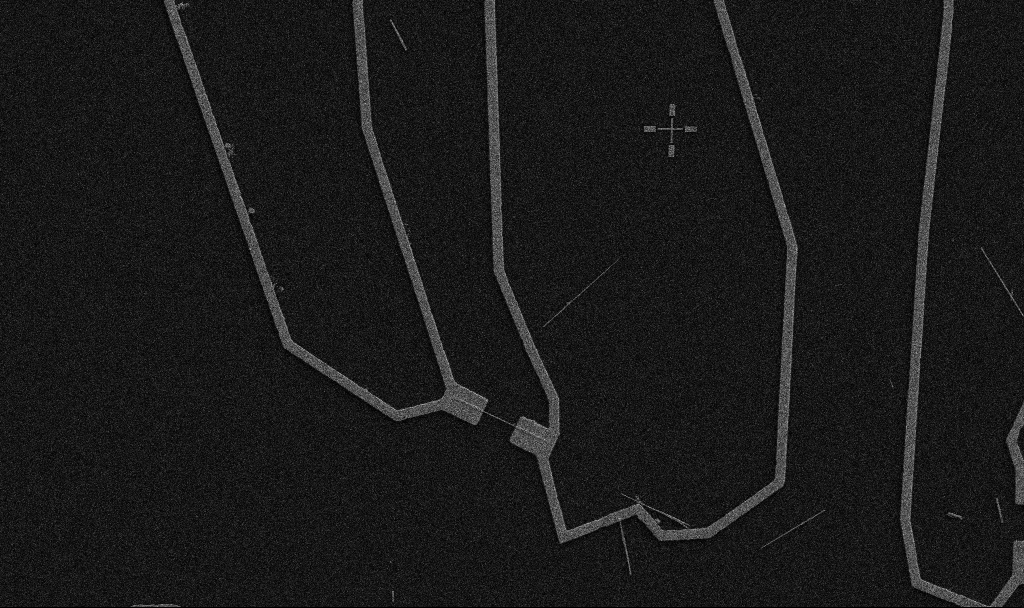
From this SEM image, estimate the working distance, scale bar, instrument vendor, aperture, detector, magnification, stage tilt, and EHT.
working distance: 10.7 mm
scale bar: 10000 nm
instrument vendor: Zeiss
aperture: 30 µm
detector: SE2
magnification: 5 K X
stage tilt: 0°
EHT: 5 kV